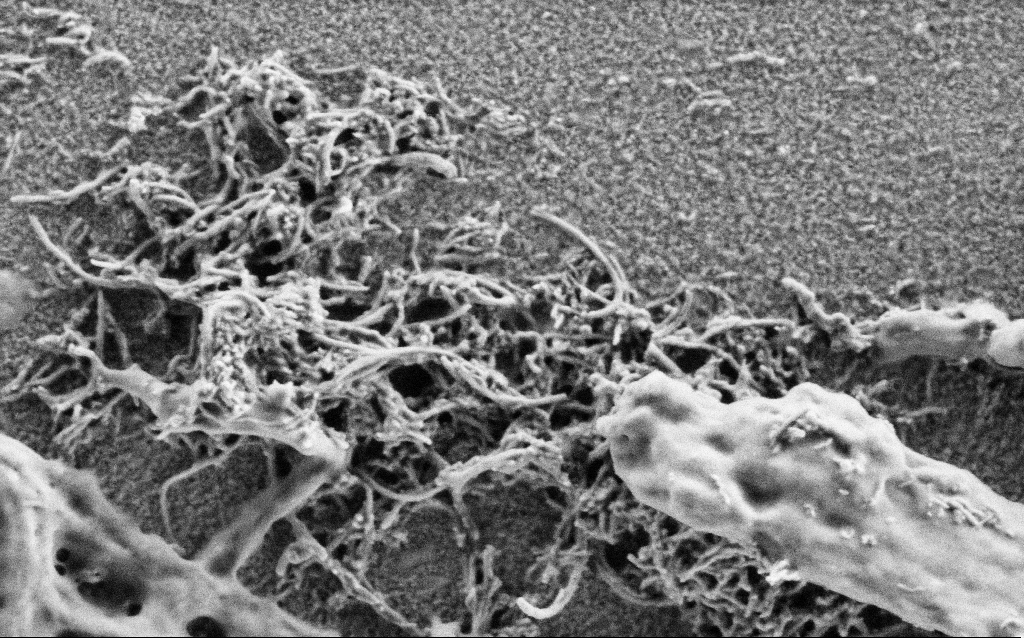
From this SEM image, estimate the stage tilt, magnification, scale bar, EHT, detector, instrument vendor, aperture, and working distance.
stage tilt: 0°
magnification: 75 K X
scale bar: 200 nm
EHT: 1 kV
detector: SE2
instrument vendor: Zeiss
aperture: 30 µm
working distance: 4 mm